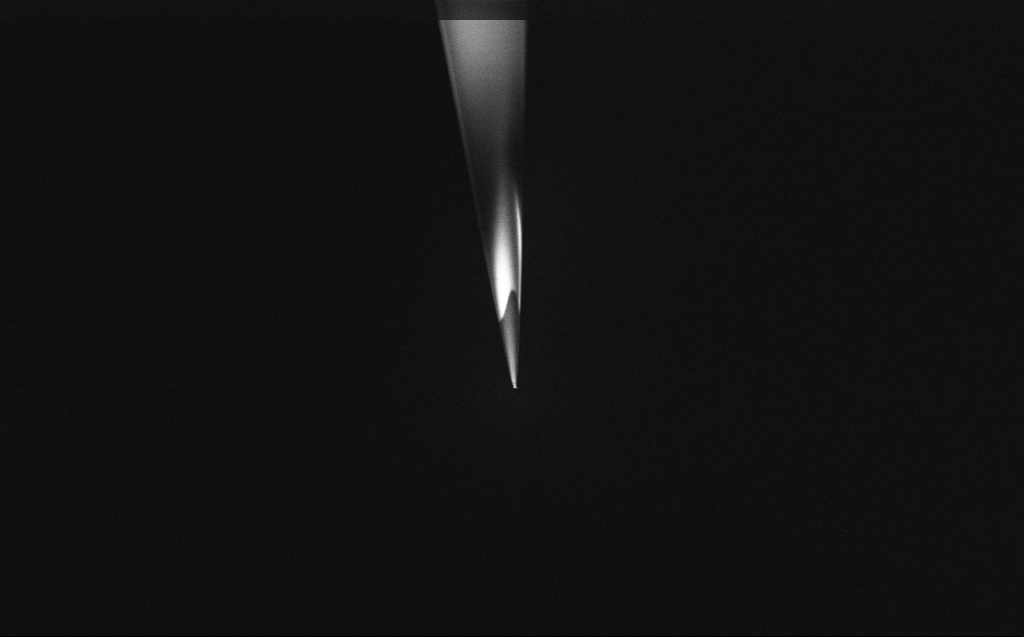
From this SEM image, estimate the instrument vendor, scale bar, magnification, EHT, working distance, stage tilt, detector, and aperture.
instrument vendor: Zeiss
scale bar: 10000 nm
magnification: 5 K X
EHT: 2 kV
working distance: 6 mm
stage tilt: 45°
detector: InLens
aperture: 30 µm